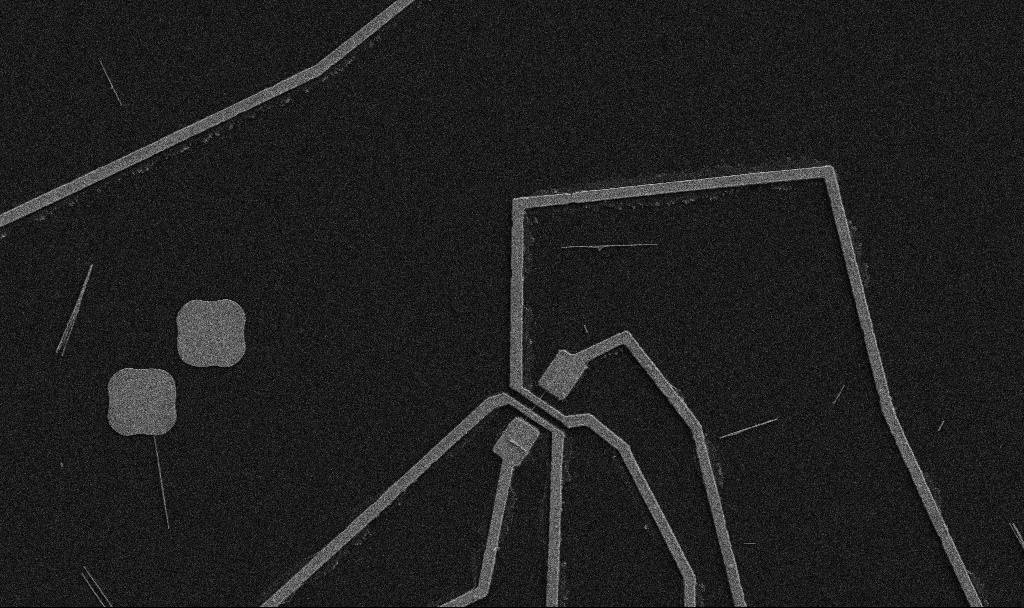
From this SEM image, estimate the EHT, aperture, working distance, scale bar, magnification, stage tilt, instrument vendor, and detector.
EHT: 5 kV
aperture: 30 µm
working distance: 10.7 mm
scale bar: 10000 nm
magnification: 5 K X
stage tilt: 0°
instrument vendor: Zeiss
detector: SE2